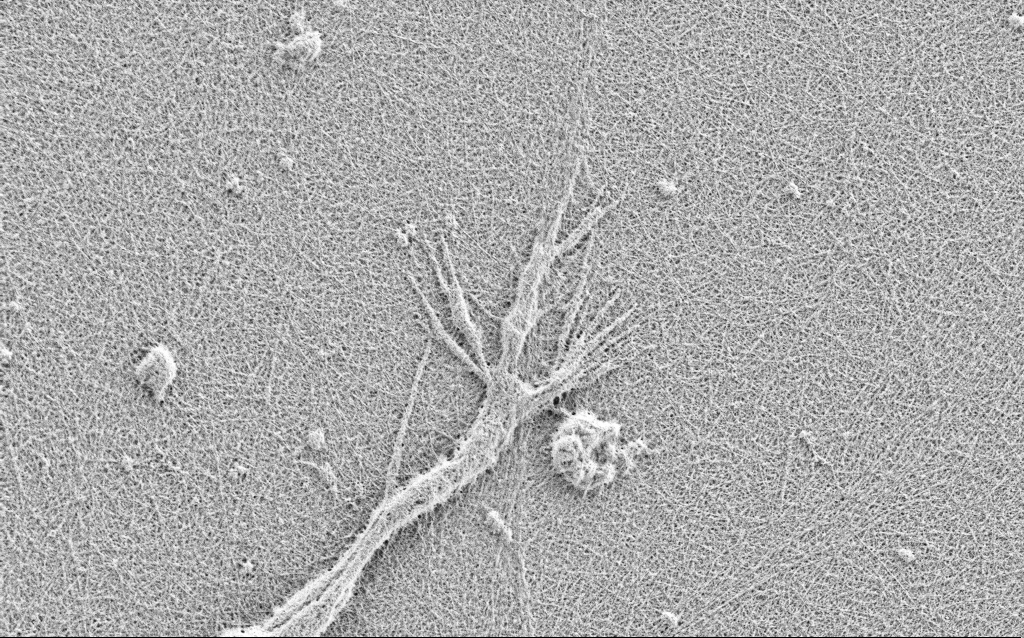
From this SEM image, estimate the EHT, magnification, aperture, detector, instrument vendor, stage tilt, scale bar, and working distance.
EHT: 0.9 kV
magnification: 10 K X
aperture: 30 µm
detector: SE2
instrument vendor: Zeiss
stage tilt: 0°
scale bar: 2000 nm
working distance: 3 mm